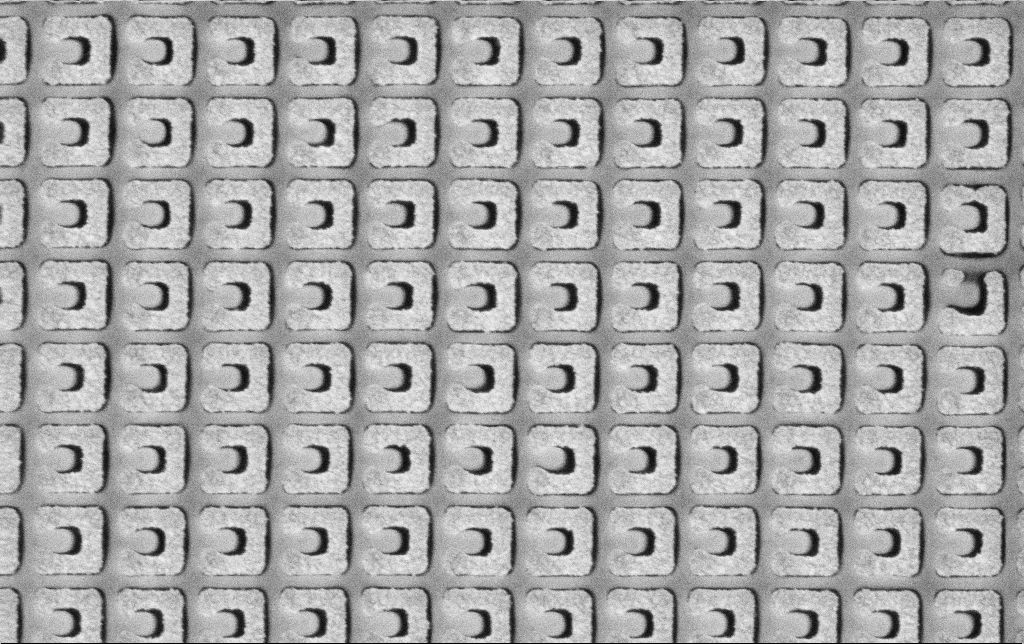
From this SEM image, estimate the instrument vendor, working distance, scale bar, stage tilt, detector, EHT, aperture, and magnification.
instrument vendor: Zeiss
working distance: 5.2 mm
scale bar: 1000 nm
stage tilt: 0°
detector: SE2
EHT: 3 kV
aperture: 30 µm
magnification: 65.31 K X